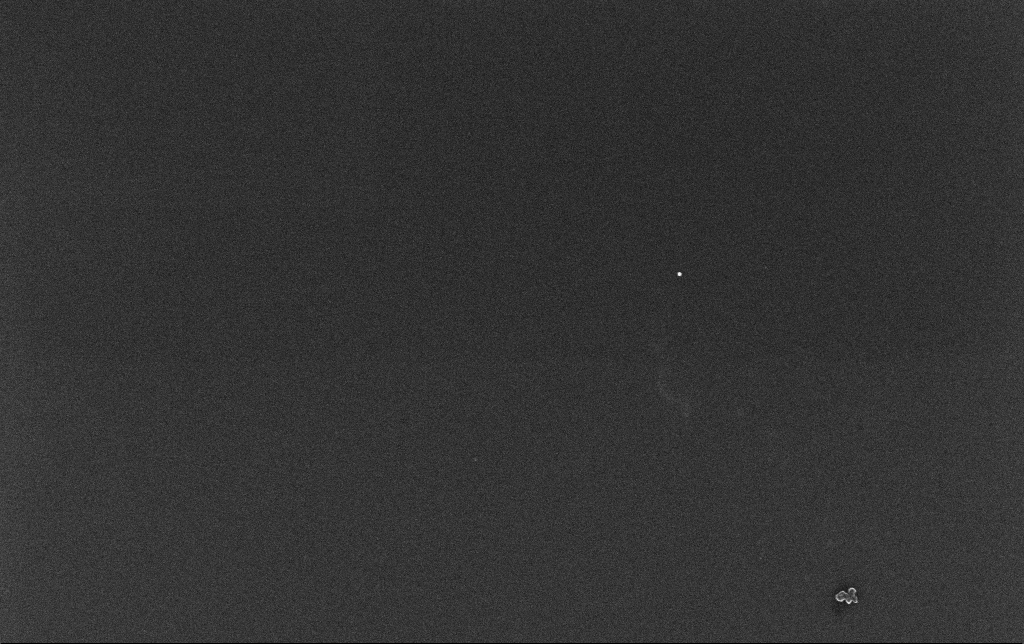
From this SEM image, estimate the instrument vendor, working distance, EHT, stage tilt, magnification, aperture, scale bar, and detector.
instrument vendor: Zeiss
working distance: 3.4 mm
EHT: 10 kV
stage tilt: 0°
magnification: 100 K X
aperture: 30 µm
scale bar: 200 nm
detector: InLens